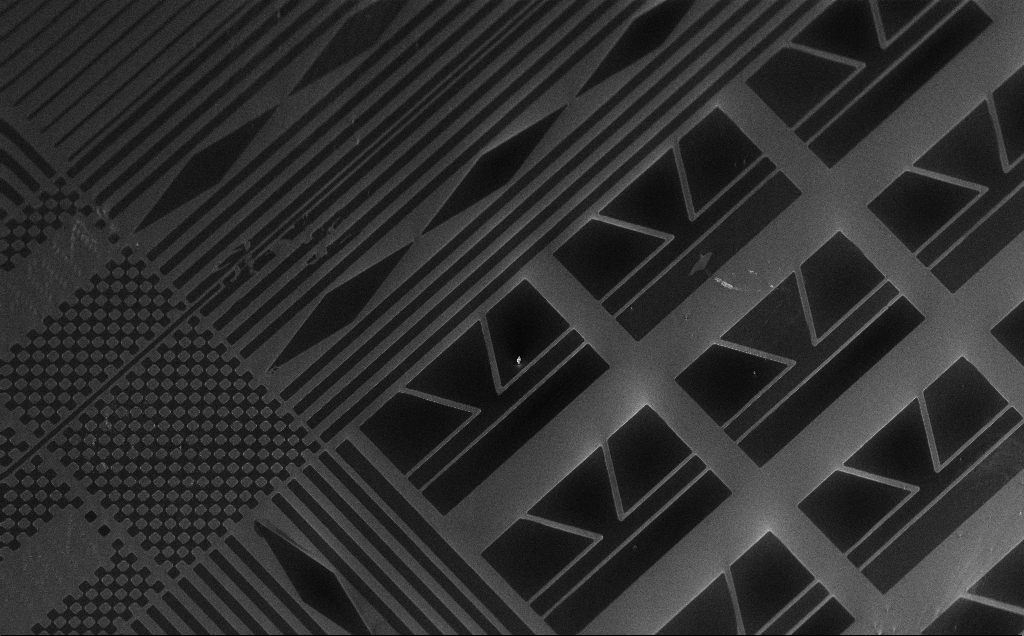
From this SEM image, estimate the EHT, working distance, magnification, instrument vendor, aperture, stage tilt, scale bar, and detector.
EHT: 10 kV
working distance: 11 mm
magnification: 0.38 K X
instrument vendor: Zeiss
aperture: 30 µm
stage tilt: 50°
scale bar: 100000 nm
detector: InLens